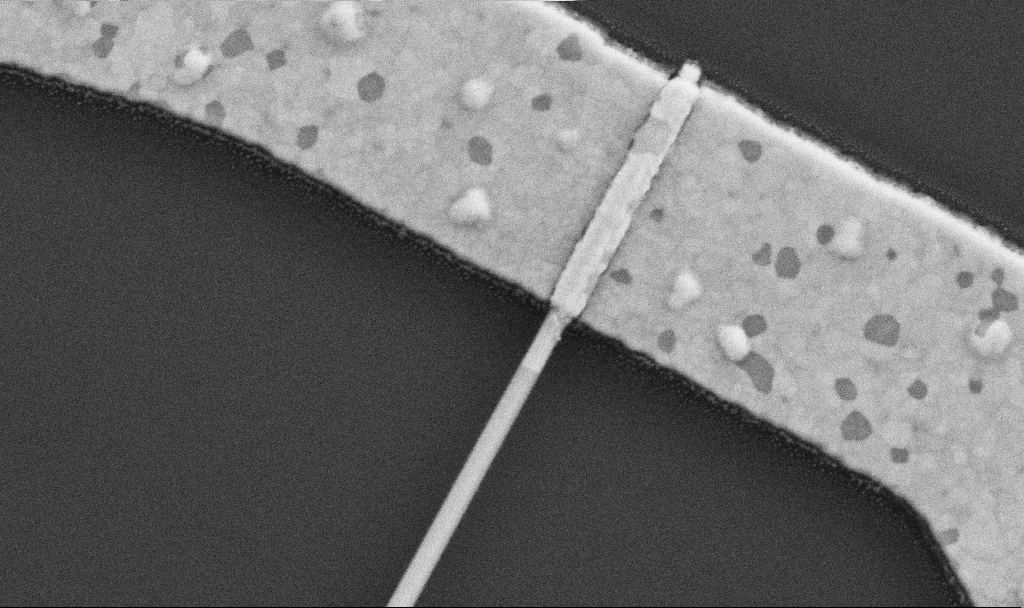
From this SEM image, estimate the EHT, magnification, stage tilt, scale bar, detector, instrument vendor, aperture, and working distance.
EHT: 5 kV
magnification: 100 K X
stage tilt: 0°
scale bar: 200 nm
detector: SE2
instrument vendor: Zeiss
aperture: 30 µm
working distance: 8.7 mm